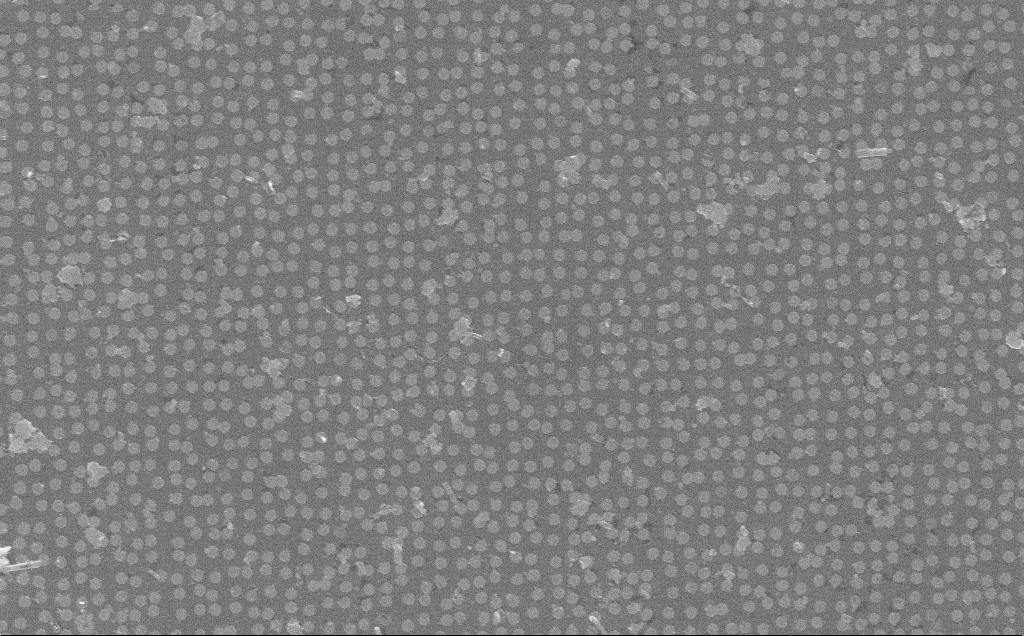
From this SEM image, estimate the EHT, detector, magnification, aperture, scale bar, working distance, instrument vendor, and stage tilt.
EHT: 10 kV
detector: InLens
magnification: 17.39 K X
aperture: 30 µm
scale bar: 2000 nm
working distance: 7 mm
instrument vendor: Zeiss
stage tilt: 0°